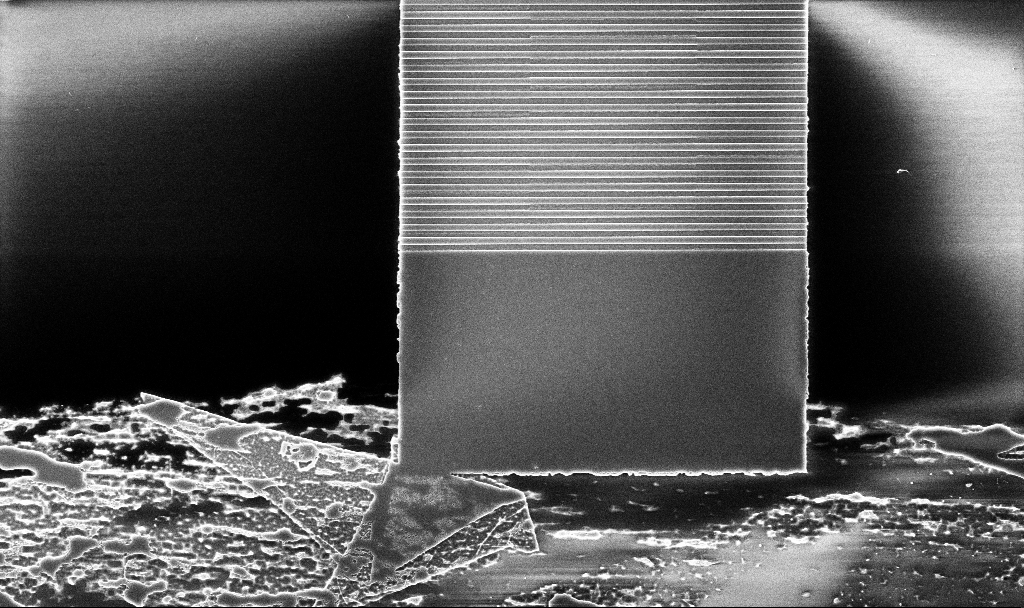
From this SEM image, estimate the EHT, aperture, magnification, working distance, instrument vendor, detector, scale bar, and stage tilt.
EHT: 5 kV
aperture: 30 µm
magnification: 7.51 K X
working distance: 10.1 mm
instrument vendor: Zeiss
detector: InLens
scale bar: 2000 nm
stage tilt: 0°